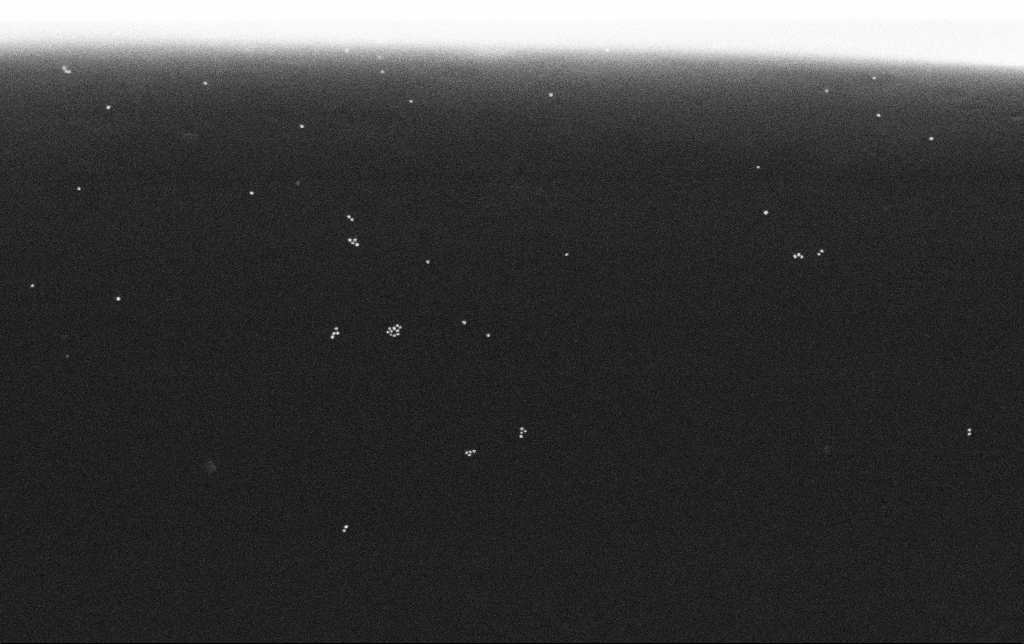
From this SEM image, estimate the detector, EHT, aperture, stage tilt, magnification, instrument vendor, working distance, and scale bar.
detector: SE2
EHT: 30 kV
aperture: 30 µm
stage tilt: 0°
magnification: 100 K X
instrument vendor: Zeiss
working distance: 10.8 mm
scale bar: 200 nm